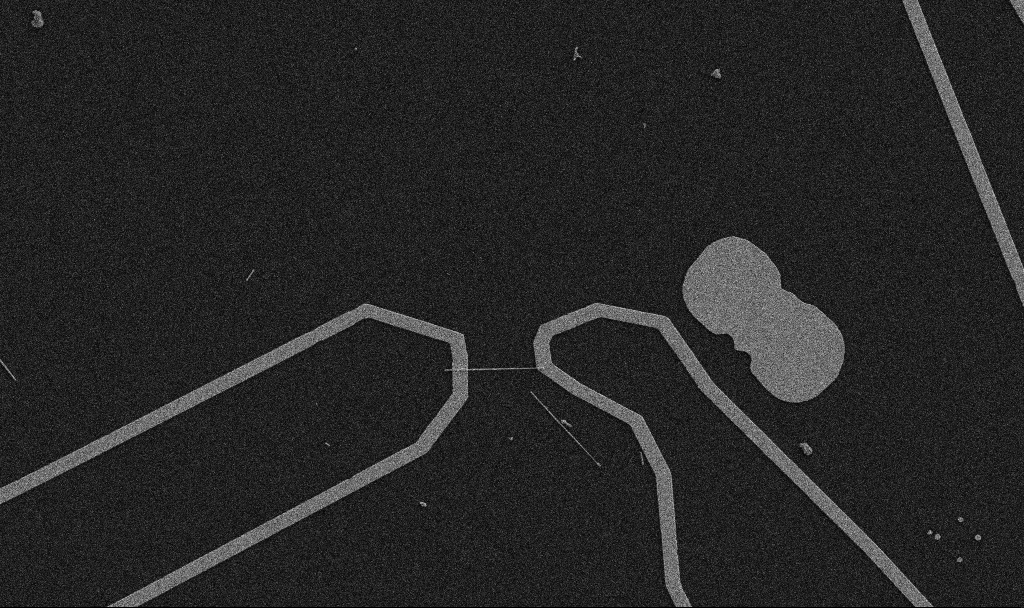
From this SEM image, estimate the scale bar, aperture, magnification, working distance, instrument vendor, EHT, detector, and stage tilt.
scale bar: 10000 nm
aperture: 30 µm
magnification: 5 K X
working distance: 10.7 mm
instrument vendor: Zeiss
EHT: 5 kV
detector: SE2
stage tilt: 0°